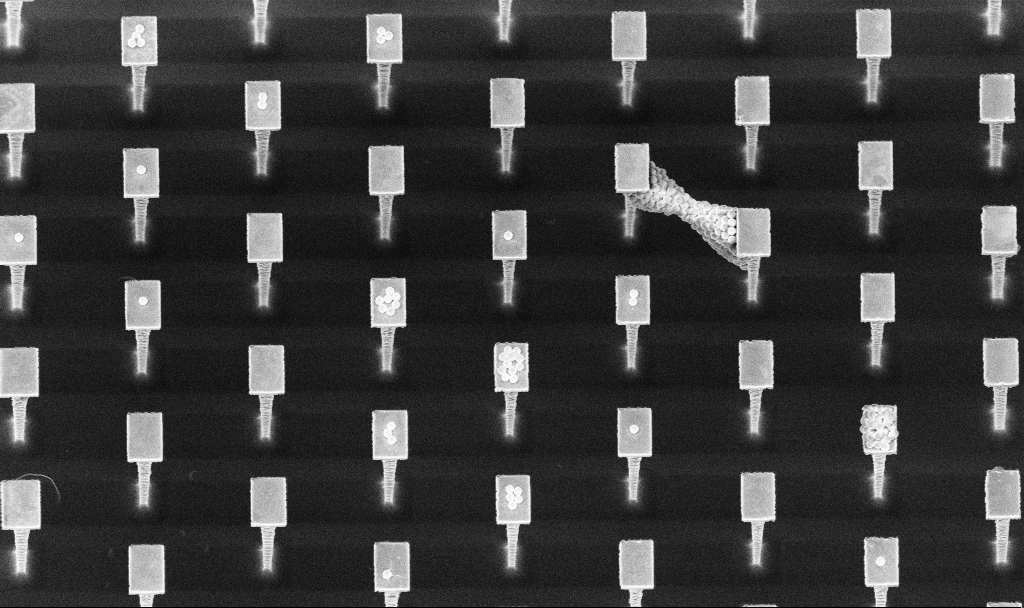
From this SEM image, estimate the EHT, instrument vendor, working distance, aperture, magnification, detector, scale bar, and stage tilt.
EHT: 5 kV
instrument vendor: Zeiss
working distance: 4.5 mm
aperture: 30 µm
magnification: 4.31 K X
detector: InLens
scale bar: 10000 nm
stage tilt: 20°